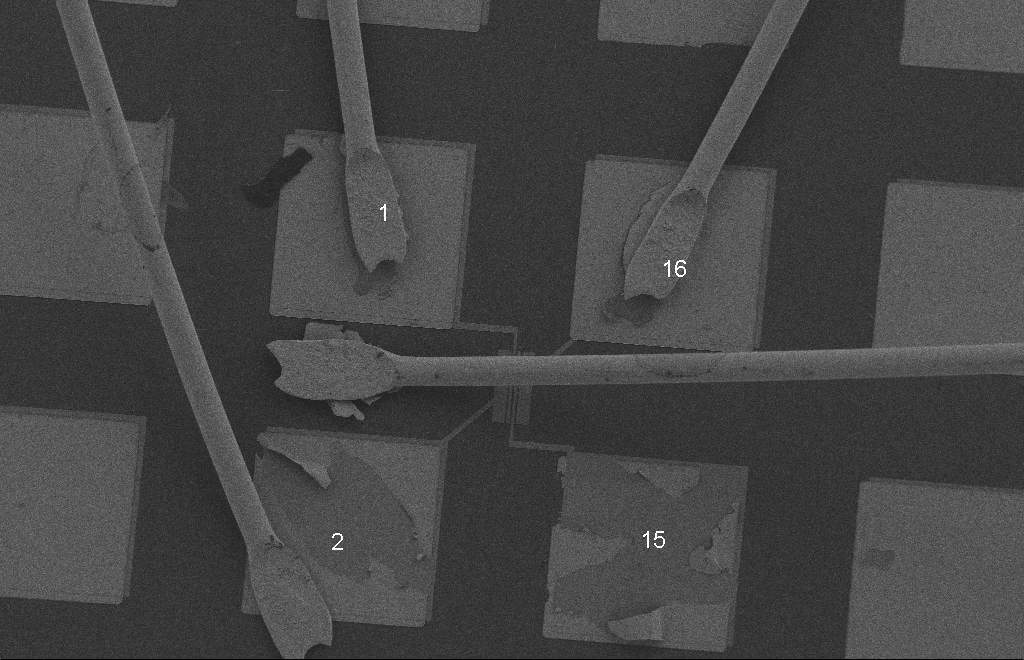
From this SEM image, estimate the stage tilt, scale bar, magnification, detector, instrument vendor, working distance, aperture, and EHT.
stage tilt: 0°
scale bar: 100000 nm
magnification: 0.44 K X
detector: SE2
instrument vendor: Zeiss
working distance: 9 mm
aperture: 20 µm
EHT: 2 kV